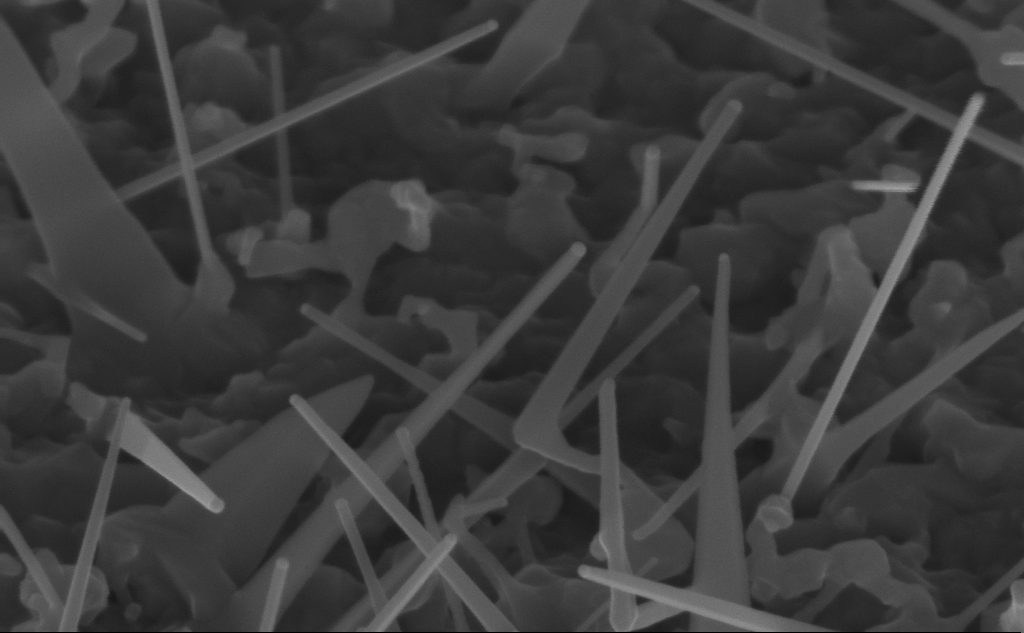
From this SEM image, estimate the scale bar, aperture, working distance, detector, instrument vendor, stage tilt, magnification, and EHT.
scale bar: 200 nm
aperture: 30 µm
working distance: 8 mm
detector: InLens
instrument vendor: Zeiss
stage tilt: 45°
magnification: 150 K X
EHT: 10 kV